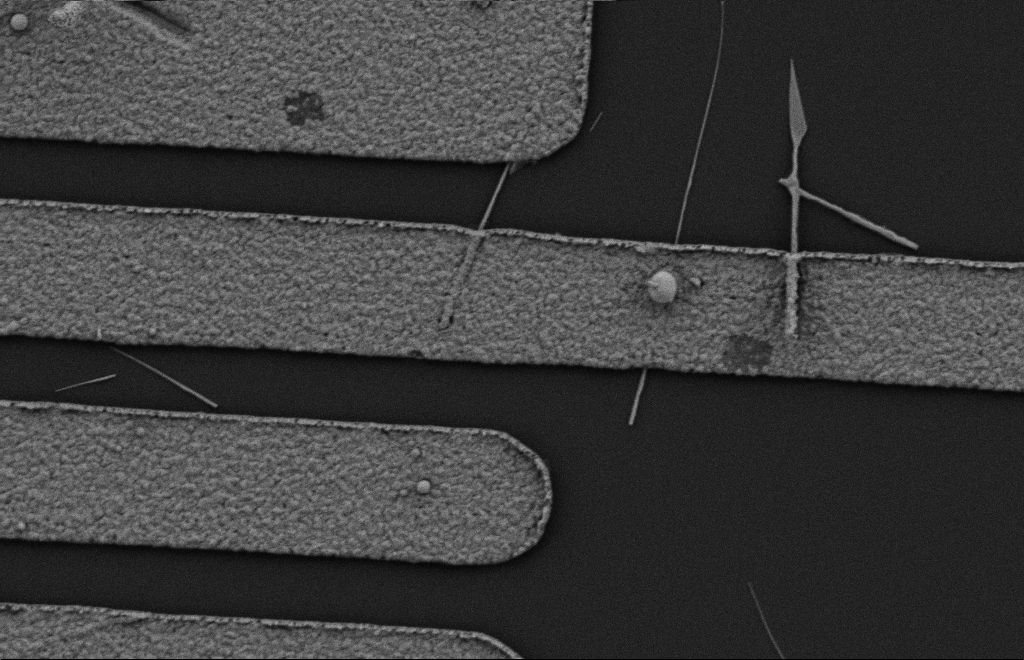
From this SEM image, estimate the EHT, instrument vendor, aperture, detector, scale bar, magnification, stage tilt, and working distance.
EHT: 2 kV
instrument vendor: Zeiss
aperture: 20 µm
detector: SE2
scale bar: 2000 nm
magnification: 18.41 K X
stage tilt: -0.3°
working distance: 10 mm